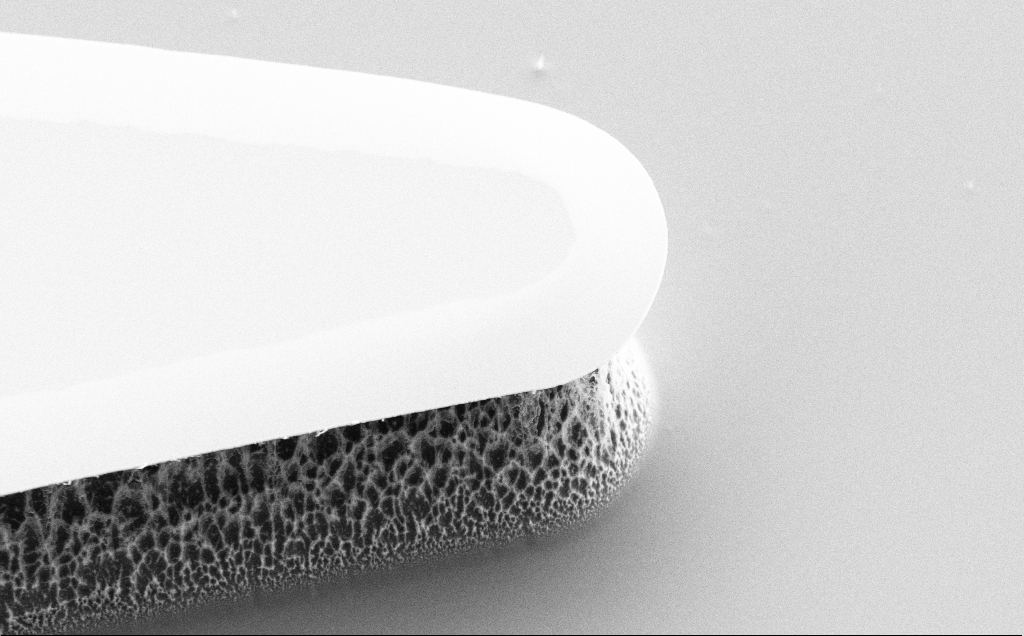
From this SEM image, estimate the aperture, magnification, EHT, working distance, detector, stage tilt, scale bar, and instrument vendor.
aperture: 30 µm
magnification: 5.38 K X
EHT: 5 kV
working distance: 7 mm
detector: SE2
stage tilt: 45°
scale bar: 10000 nm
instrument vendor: Zeiss